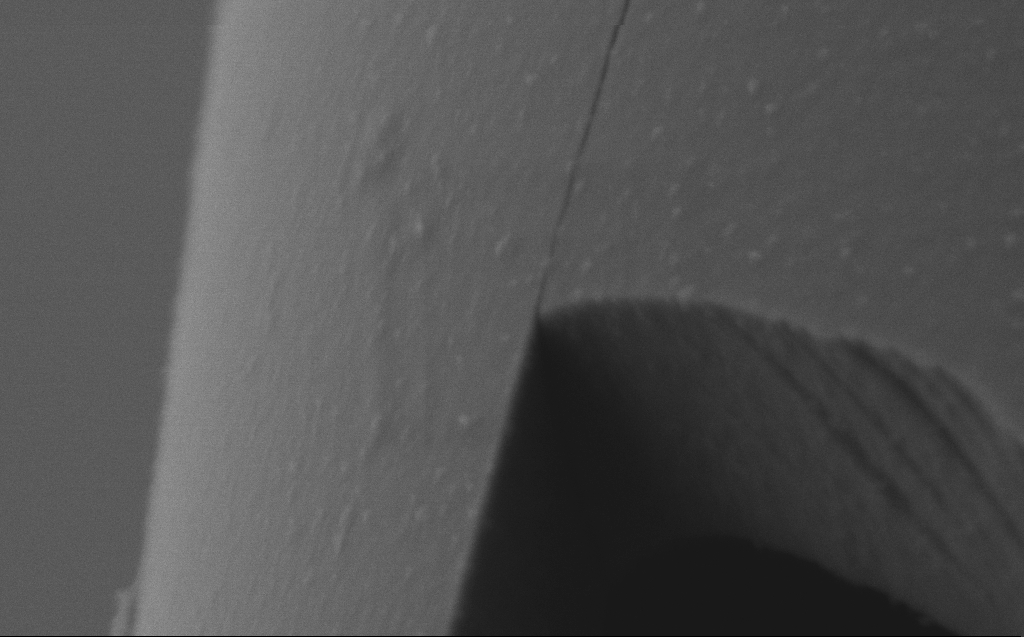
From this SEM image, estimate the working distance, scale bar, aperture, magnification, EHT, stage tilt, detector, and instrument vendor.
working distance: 5 mm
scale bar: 200 nm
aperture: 30 µm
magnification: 147.43 K X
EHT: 2 kV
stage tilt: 45°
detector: SE2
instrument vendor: Zeiss